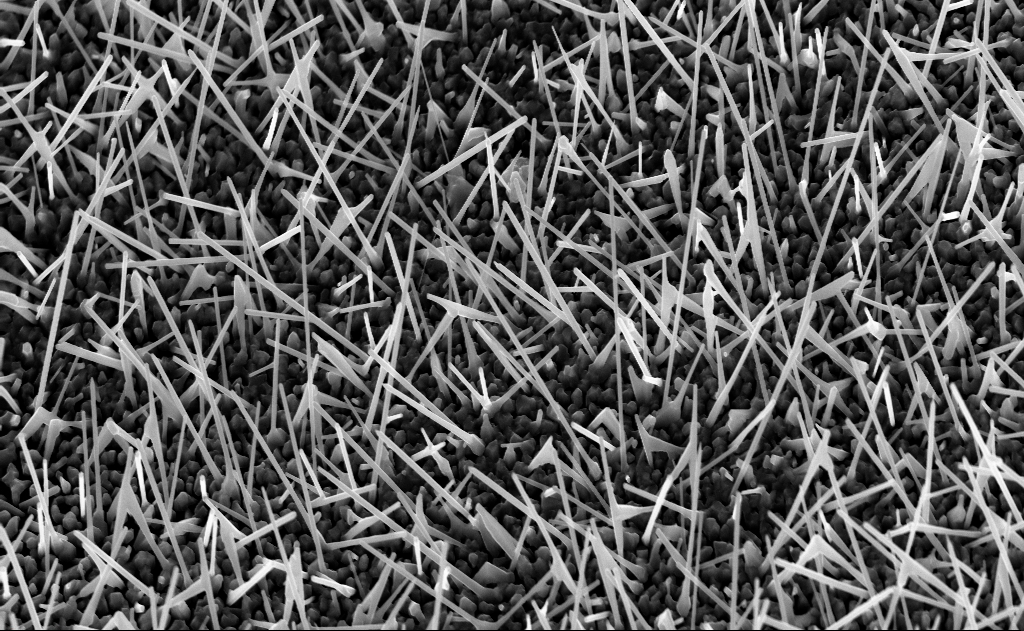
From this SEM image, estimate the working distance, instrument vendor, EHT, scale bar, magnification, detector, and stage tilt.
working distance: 6 mm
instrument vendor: Zeiss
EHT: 10 kV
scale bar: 2000 nm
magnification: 20 K X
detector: InLens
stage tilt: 0°